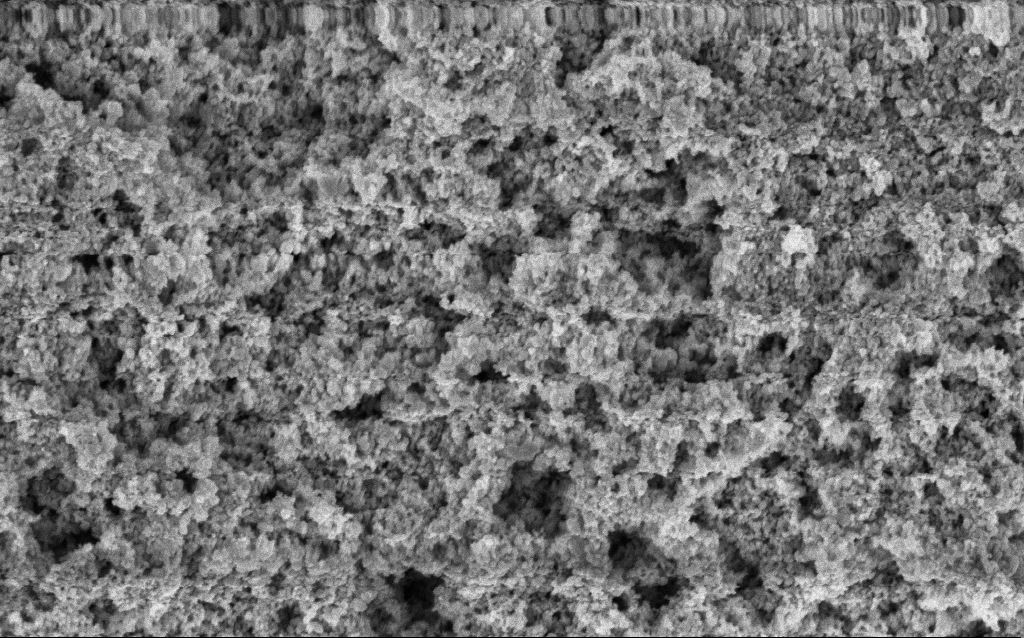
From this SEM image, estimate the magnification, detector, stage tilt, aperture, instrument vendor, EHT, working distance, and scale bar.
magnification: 65.04 K X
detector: InLens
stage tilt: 0°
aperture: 30 µm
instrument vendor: Zeiss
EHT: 3 kV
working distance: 10 mm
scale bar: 1000 nm